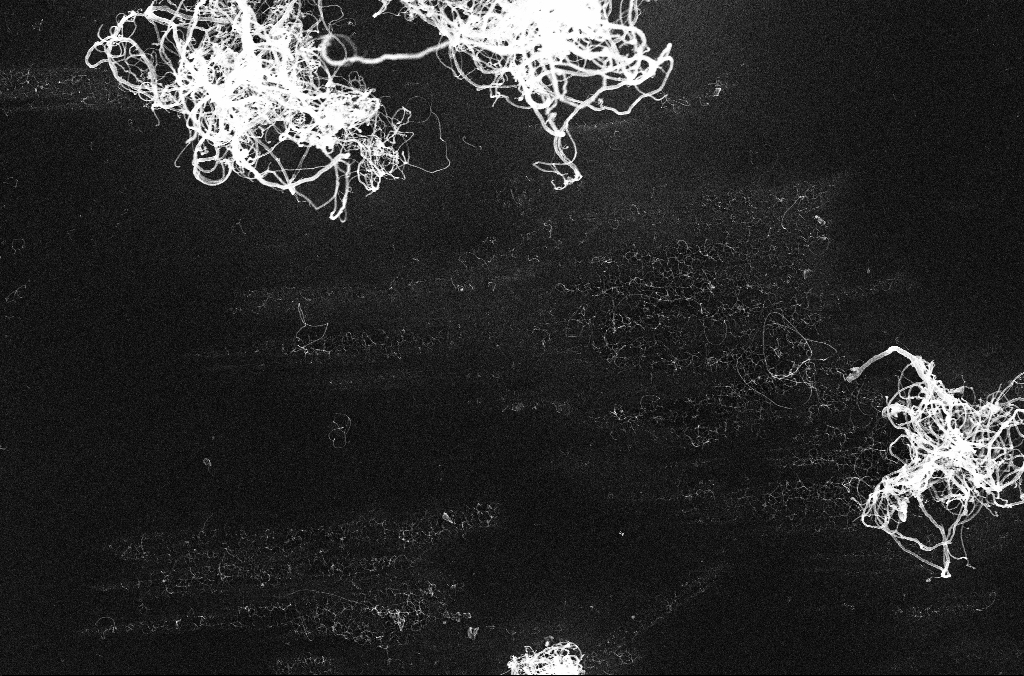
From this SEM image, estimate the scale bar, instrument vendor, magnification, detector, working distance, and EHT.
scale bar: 1000 nm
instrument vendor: Zeiss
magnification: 15 K X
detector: InLens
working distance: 3.3 mm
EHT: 10 kV